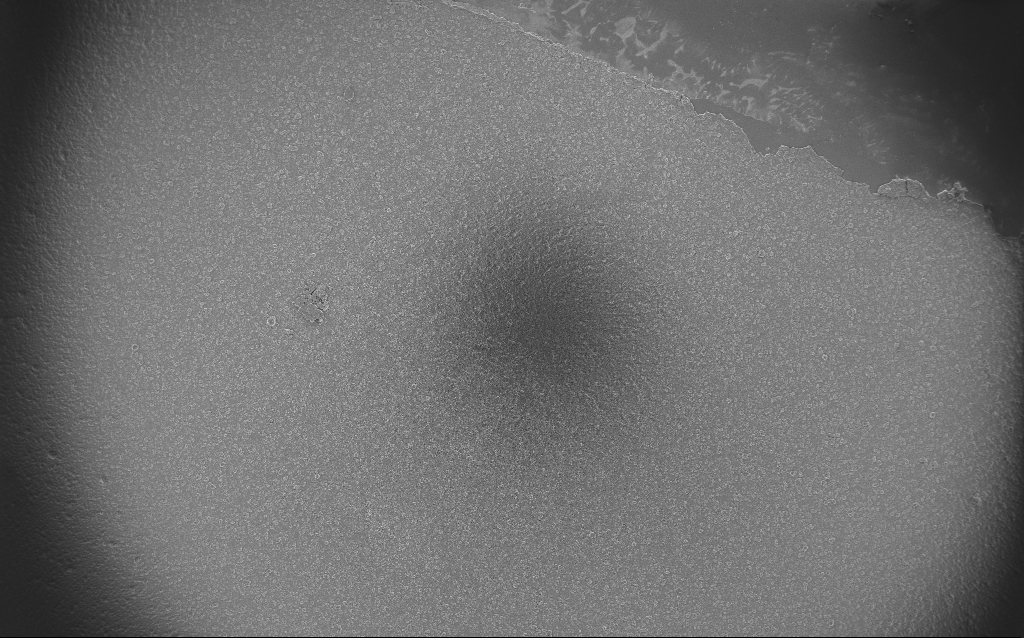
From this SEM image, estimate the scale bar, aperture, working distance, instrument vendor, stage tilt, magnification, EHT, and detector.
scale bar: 200000 nm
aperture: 20 µm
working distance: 2.6 mm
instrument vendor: Zeiss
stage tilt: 0°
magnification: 0.11 K X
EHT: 5 kV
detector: InLens